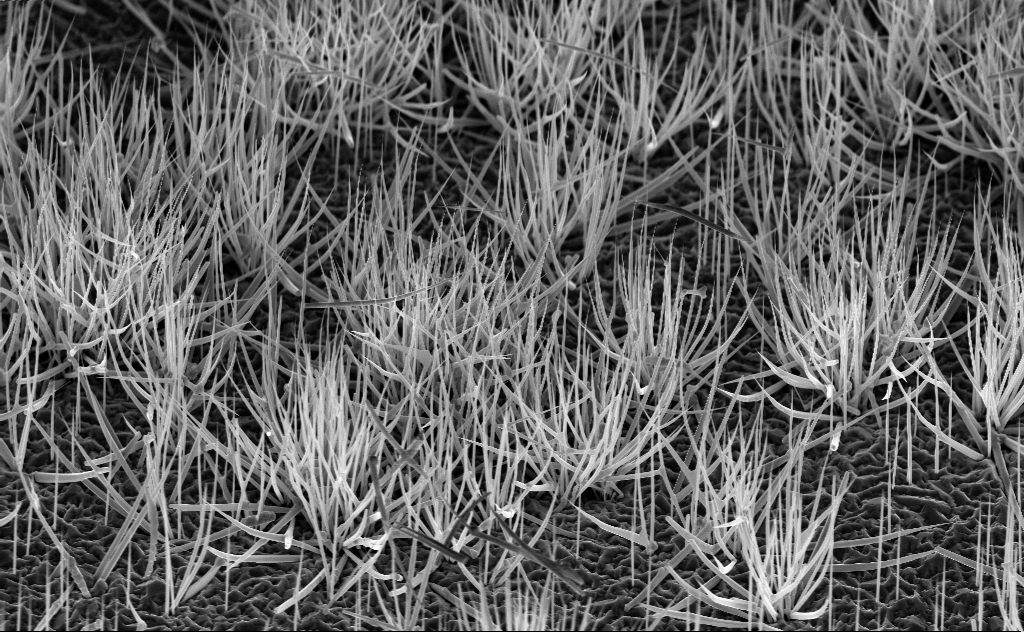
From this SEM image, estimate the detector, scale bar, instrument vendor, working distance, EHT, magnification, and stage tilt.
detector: InLens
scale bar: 10000 nm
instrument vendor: Zeiss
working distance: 7 mm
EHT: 10 kV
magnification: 5.68 K X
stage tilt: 45°